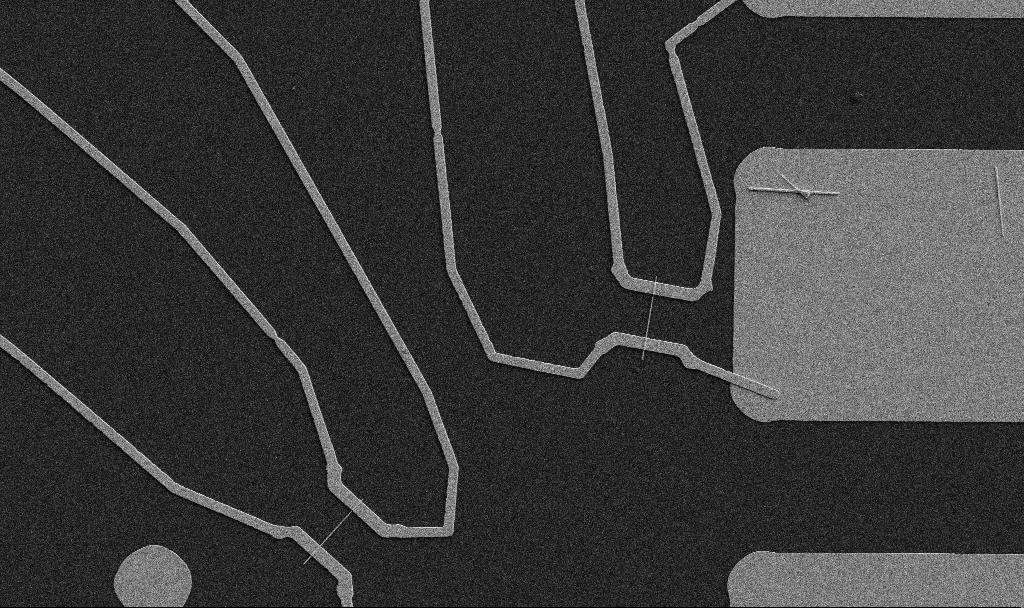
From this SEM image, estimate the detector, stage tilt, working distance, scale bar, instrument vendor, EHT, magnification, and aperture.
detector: SE2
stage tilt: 0°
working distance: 10.7 mm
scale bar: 10000 nm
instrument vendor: Zeiss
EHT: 5 kV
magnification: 5 K X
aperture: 30 µm